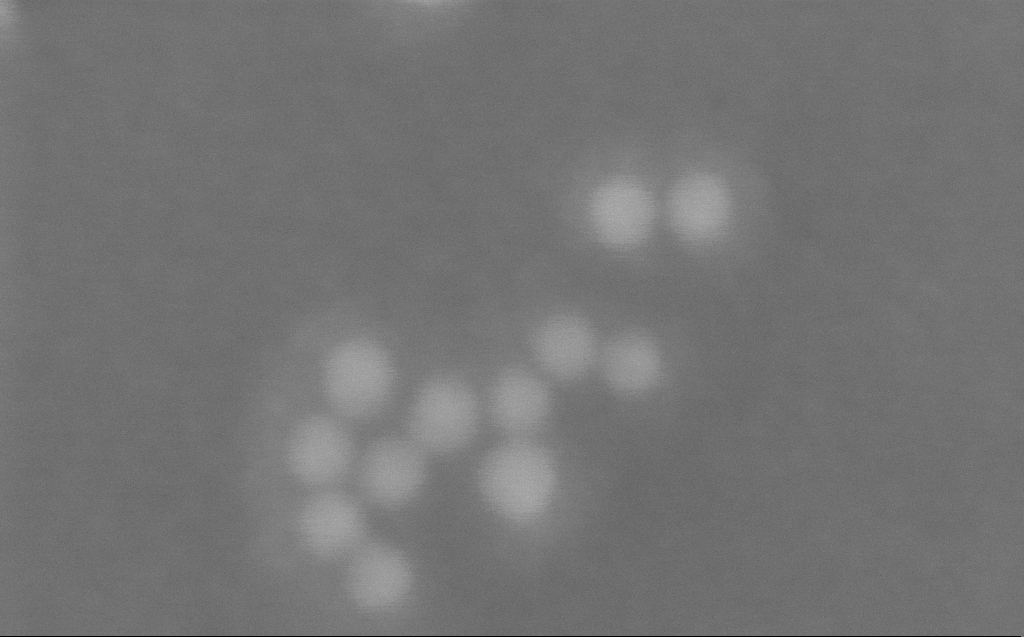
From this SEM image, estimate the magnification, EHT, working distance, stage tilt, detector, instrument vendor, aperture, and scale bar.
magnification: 1628.87 K X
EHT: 10 kV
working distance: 3 mm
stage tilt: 0°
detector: InLens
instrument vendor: Zeiss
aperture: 30 µm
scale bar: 20 nm